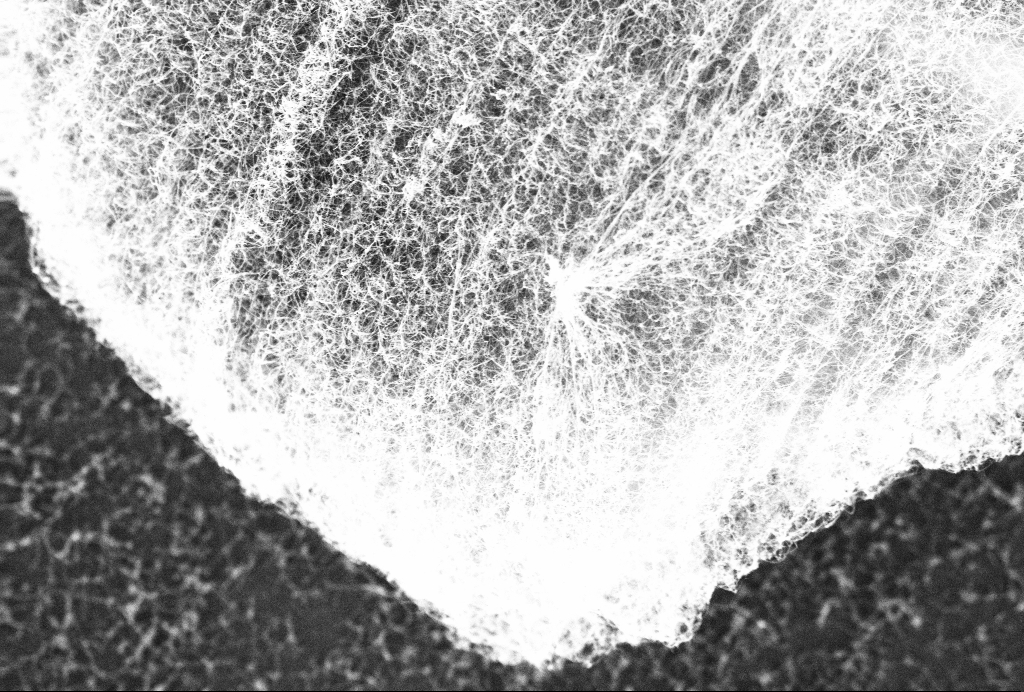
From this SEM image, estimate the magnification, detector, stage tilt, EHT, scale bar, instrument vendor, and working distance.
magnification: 46.53 K X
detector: InLens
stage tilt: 0°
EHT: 10 kV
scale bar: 1000 nm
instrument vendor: Zeiss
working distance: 2.7 mm